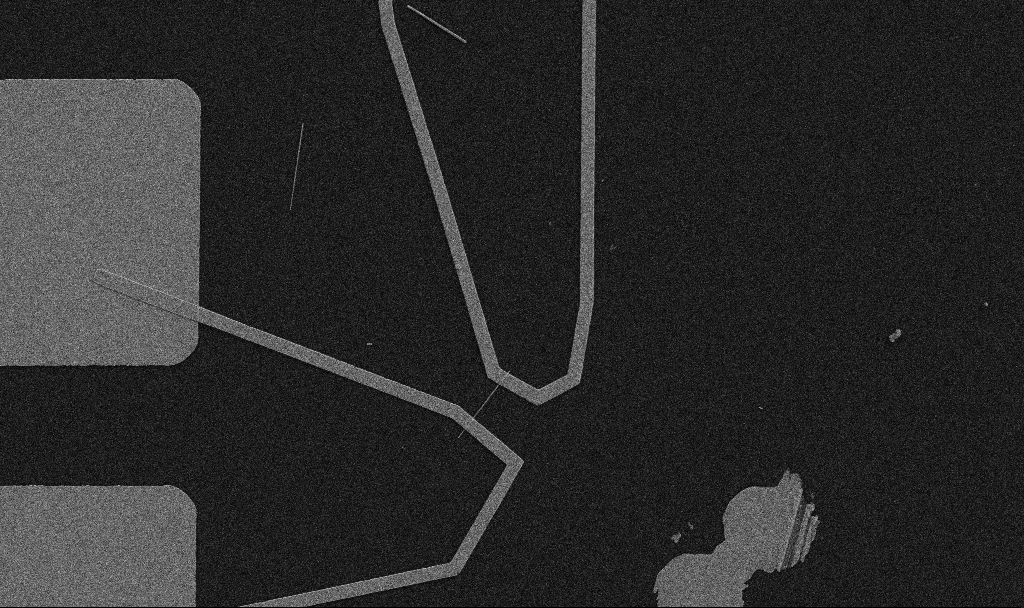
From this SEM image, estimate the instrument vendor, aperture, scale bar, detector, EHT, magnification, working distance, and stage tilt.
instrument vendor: Zeiss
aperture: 30 µm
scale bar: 10000 nm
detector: SE2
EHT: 5 kV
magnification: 5 K X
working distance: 10.7 mm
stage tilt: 0°